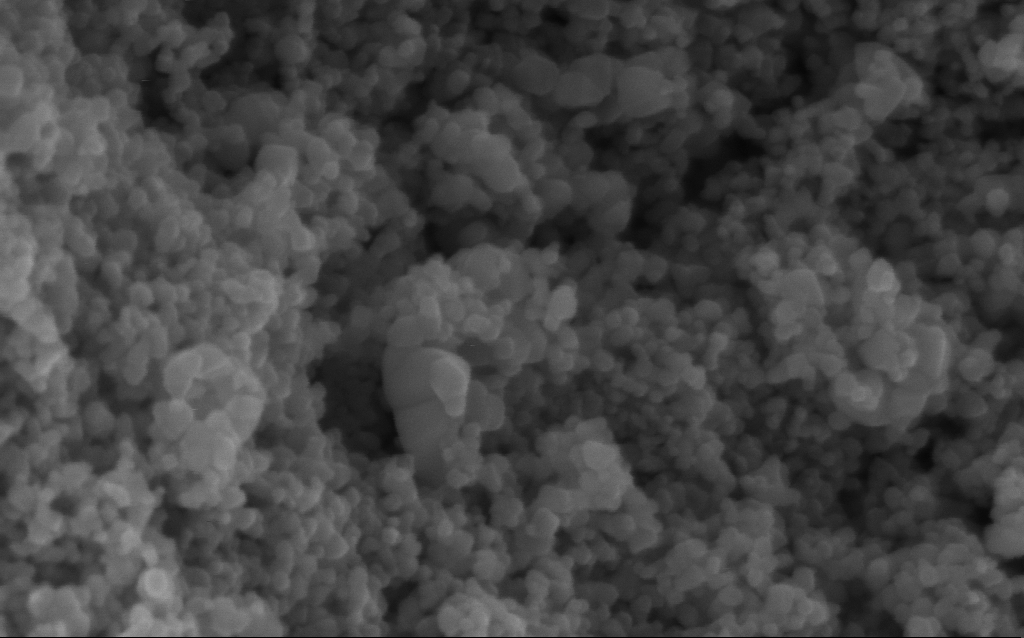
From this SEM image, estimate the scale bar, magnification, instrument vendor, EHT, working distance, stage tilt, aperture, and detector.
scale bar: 100 nm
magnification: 281.76 K X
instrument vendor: Zeiss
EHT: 5 kV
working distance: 4.6 mm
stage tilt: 0°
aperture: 30 µm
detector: InLens